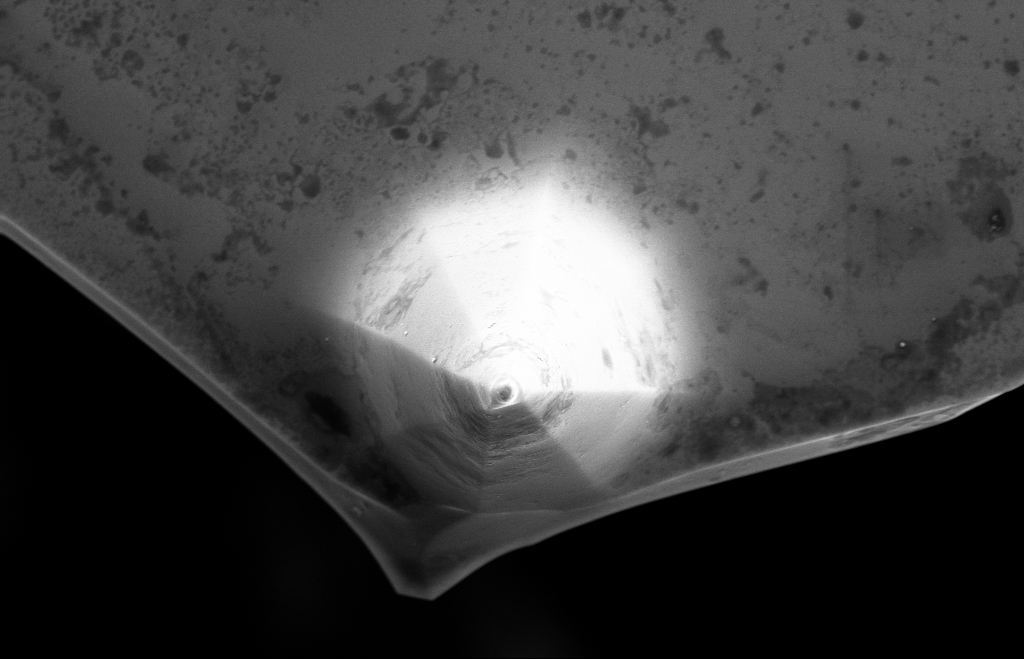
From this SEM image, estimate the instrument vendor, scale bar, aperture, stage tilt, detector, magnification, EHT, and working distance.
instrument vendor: Zeiss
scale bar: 2000 nm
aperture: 30 µm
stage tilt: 0°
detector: InLens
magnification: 10.66 K X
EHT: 10 kV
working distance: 8 mm